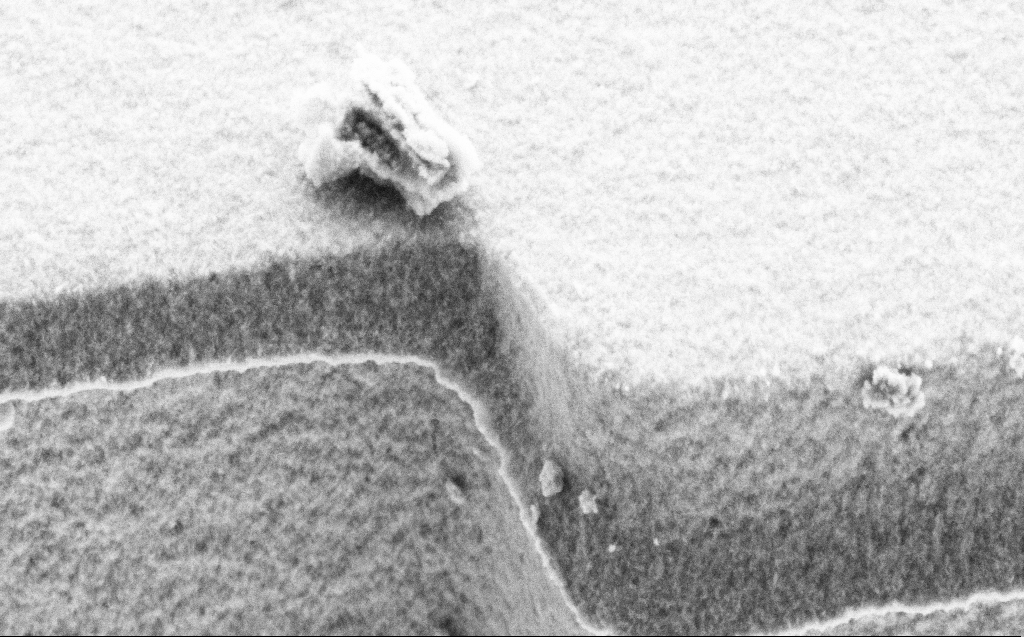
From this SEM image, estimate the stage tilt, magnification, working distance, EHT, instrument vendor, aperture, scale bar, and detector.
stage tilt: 45°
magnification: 50.24 K X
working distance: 4 mm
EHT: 3 kV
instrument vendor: Zeiss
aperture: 30 µm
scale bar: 1000 nm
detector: SE2